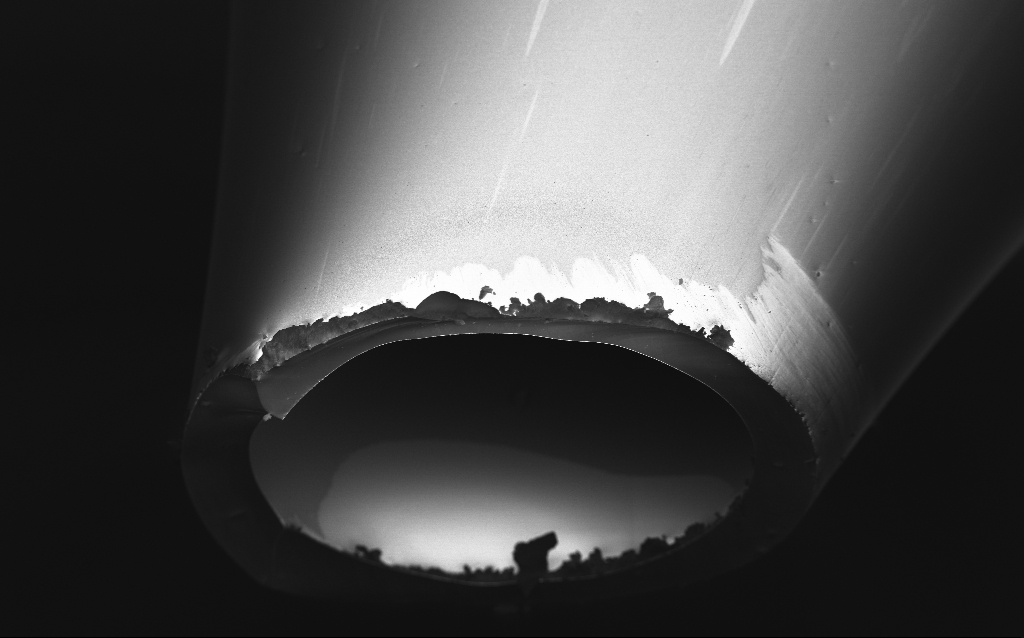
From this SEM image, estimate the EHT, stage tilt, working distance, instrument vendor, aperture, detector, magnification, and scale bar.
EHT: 1 kV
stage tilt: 45°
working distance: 5 mm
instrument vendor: Zeiss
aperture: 30 µm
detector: InLens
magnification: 0.652 K X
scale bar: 100000 nm